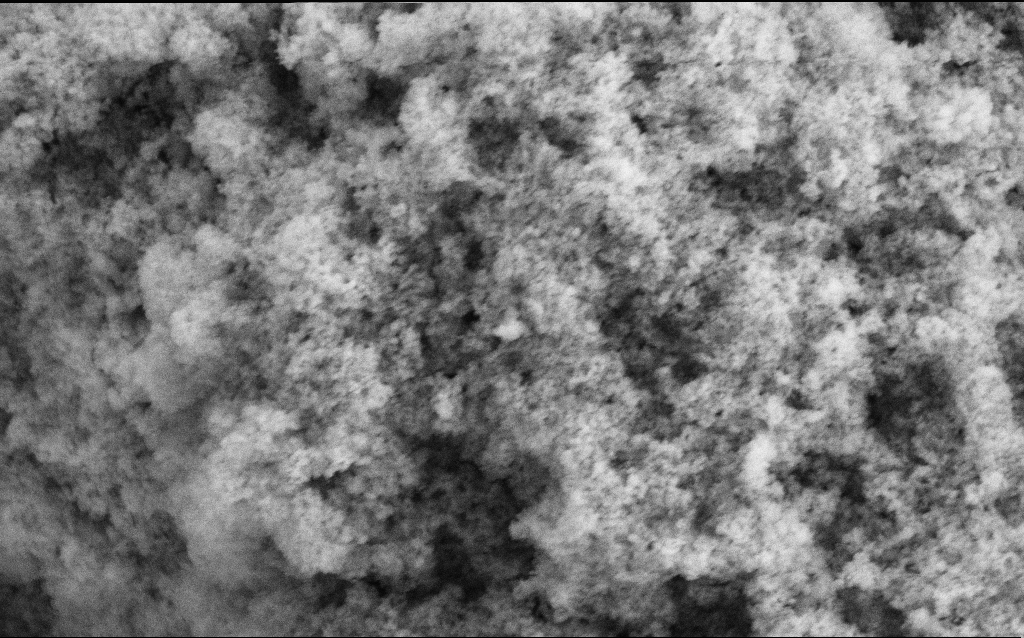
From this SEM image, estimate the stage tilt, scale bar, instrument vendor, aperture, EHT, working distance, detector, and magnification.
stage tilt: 0°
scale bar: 1000 nm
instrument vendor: Zeiss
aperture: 30 µm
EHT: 5 kV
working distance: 4.6 mm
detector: SE2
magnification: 68.64 K X